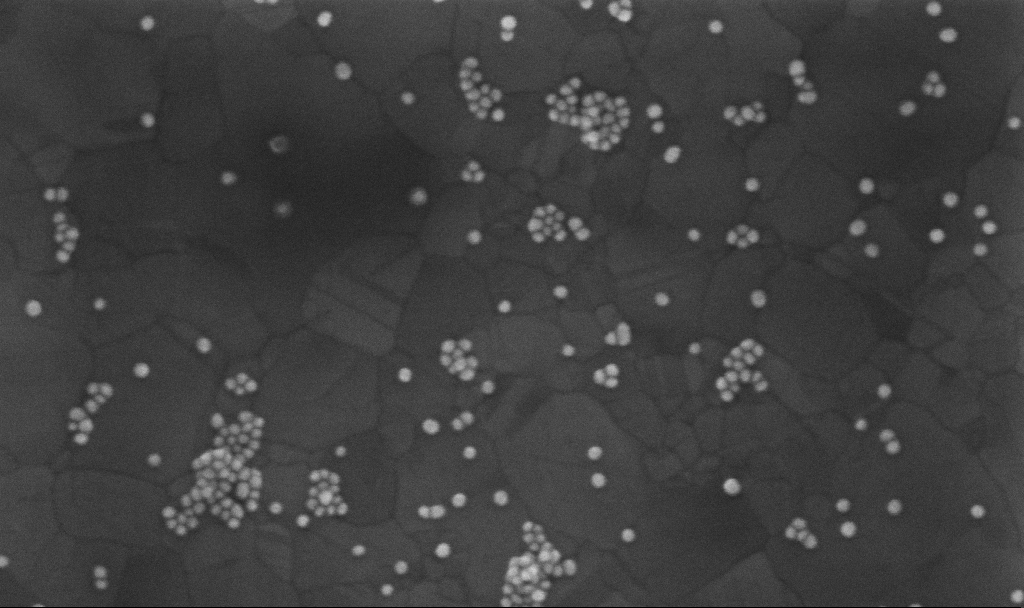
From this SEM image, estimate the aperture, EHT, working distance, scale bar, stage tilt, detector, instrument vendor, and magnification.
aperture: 30 µm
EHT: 10 kV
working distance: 3.8 mm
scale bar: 100 nm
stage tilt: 0°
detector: InLens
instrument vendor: Zeiss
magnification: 250 K X